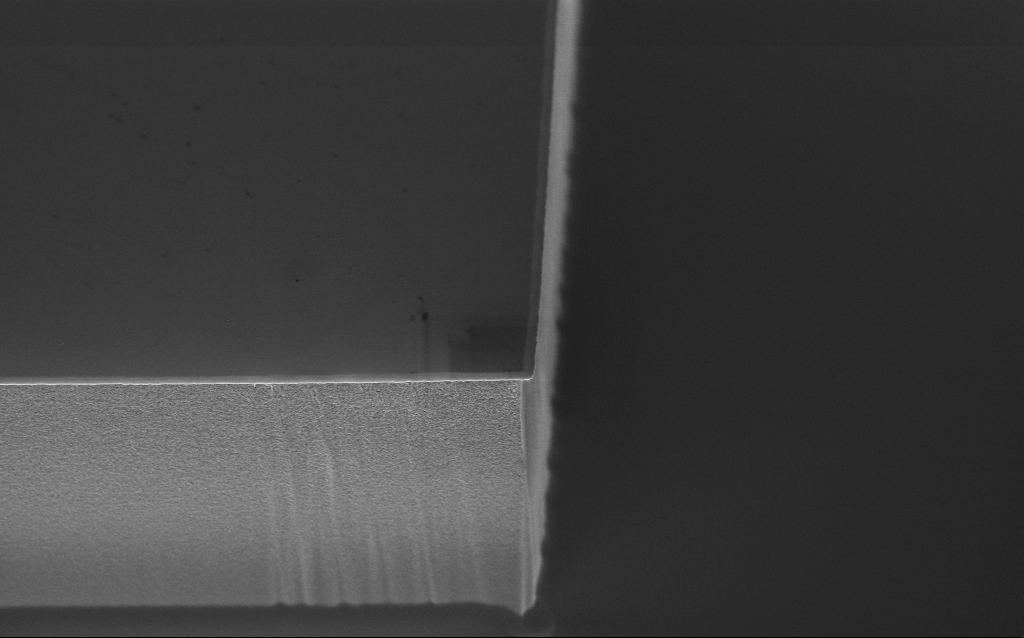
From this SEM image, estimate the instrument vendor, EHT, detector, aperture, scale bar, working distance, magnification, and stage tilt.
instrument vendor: Zeiss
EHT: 3 kV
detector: InLens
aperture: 30 µm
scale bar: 2000 nm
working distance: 6.2 mm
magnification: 11.49 K X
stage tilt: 45°